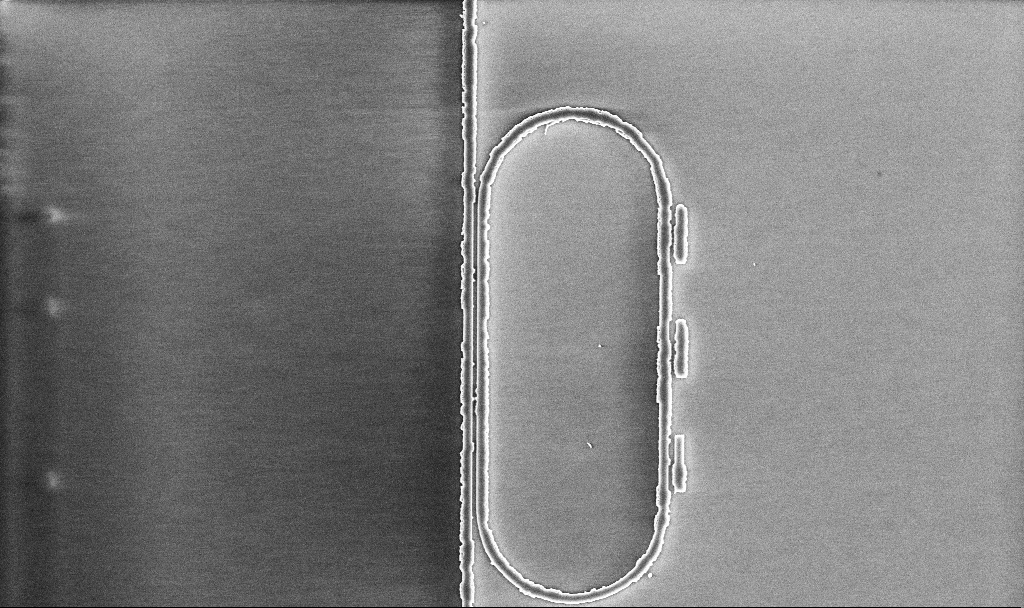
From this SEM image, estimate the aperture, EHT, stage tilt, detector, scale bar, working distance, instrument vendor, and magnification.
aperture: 30 µm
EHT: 5 kV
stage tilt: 0°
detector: InLens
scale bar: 10000 nm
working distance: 10.1 mm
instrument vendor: Zeiss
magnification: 7.11 K X